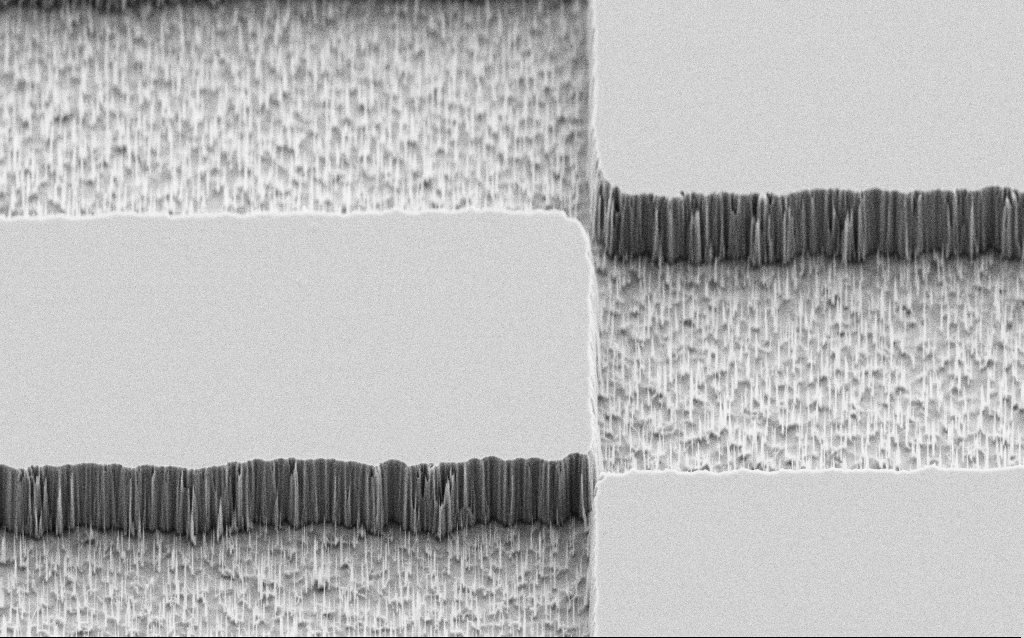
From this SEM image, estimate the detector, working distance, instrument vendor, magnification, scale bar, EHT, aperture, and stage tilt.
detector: SE2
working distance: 7 mm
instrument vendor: Zeiss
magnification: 5 K X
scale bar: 10000 nm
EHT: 5 kV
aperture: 30 µm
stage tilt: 45°